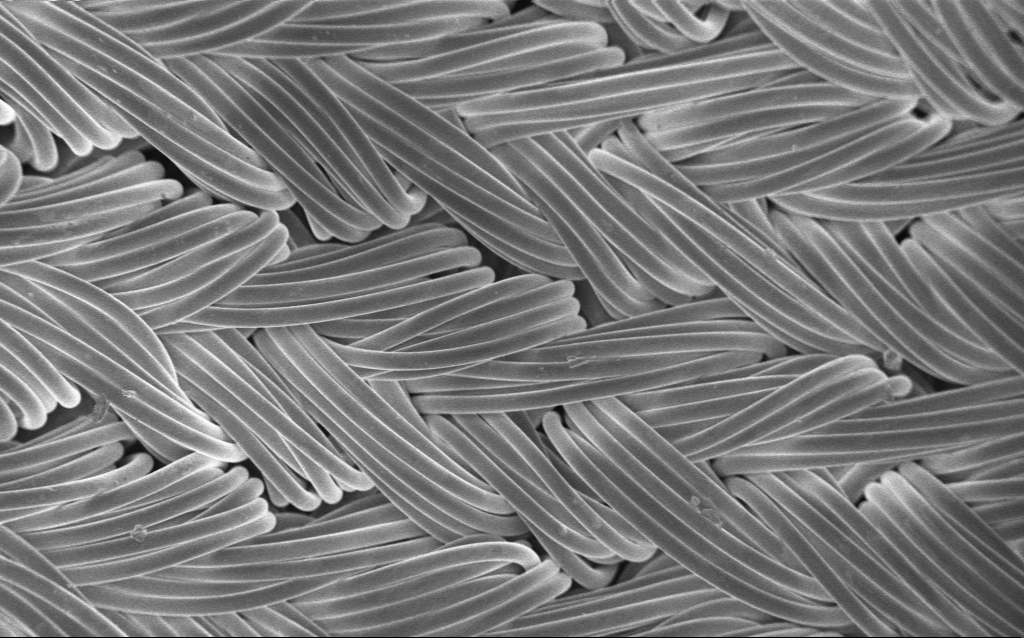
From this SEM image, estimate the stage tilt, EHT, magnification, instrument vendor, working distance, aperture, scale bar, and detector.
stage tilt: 0°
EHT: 1 kV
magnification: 0.29 K X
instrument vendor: Zeiss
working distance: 4 mm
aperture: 30 µm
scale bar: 200000 nm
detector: InLens